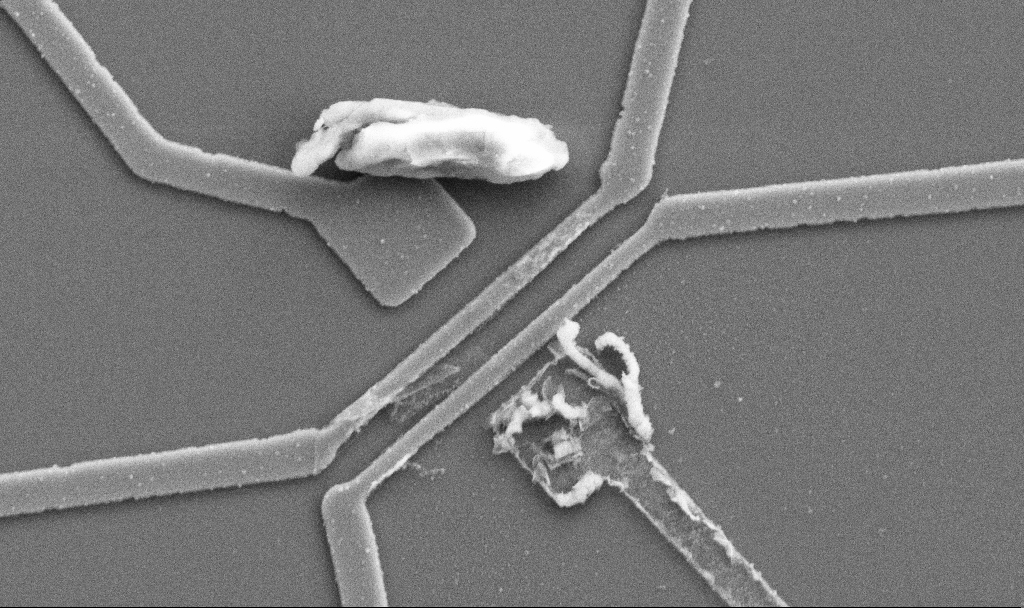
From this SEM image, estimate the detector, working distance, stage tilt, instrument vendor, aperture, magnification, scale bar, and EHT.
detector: SE2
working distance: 10.7 mm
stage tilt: -0°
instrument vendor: Zeiss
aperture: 30 µm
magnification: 20 K X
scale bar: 1000 nm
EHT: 5 kV